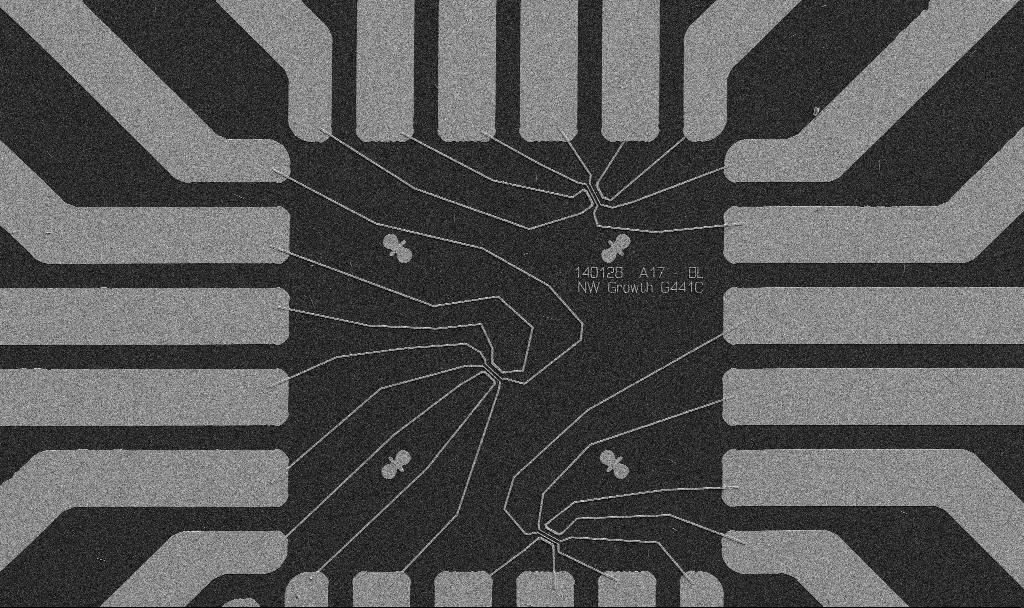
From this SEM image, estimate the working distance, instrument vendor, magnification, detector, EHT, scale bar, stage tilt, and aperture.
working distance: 10.7 mm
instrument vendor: Zeiss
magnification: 1 K X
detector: SE2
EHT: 5 kV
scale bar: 20000 nm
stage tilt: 0°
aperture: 30 µm